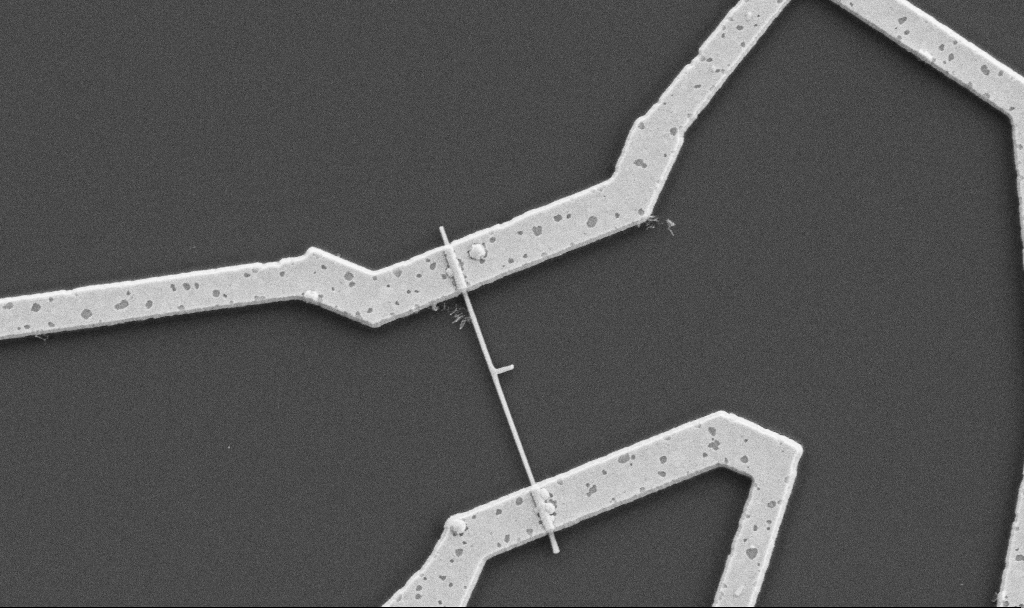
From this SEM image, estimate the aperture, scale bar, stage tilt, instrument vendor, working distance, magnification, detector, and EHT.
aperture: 30 µm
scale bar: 1000 nm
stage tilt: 0°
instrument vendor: Zeiss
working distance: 10.7 mm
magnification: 20 K X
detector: SE2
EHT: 5 kV